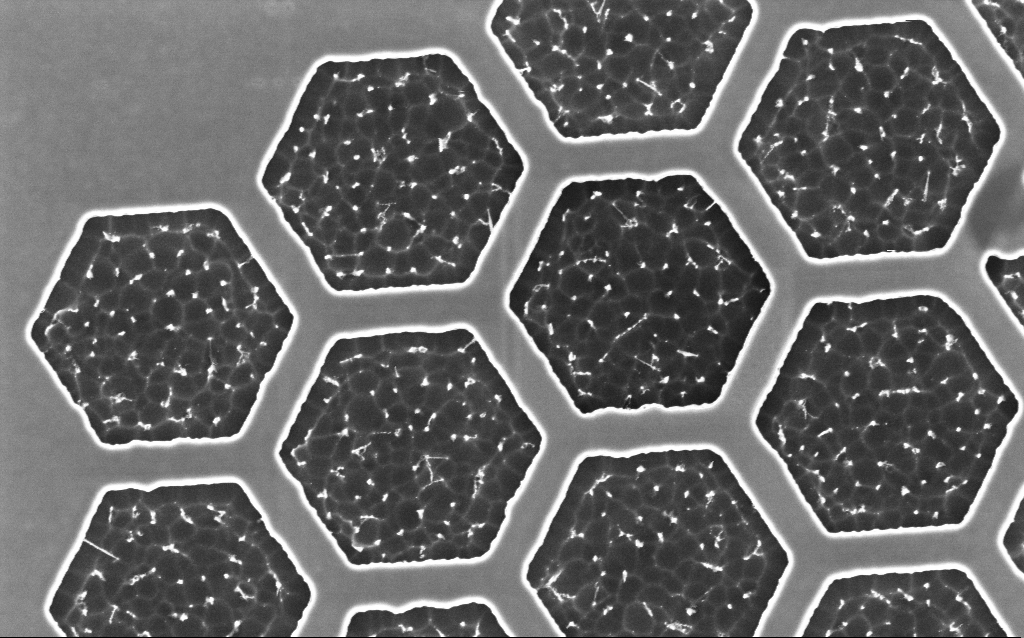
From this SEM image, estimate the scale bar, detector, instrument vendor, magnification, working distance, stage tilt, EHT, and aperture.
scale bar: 1000 nm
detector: InLens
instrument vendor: Zeiss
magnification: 32.69 K X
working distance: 5 mm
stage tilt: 0°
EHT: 2 kV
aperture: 30 µm